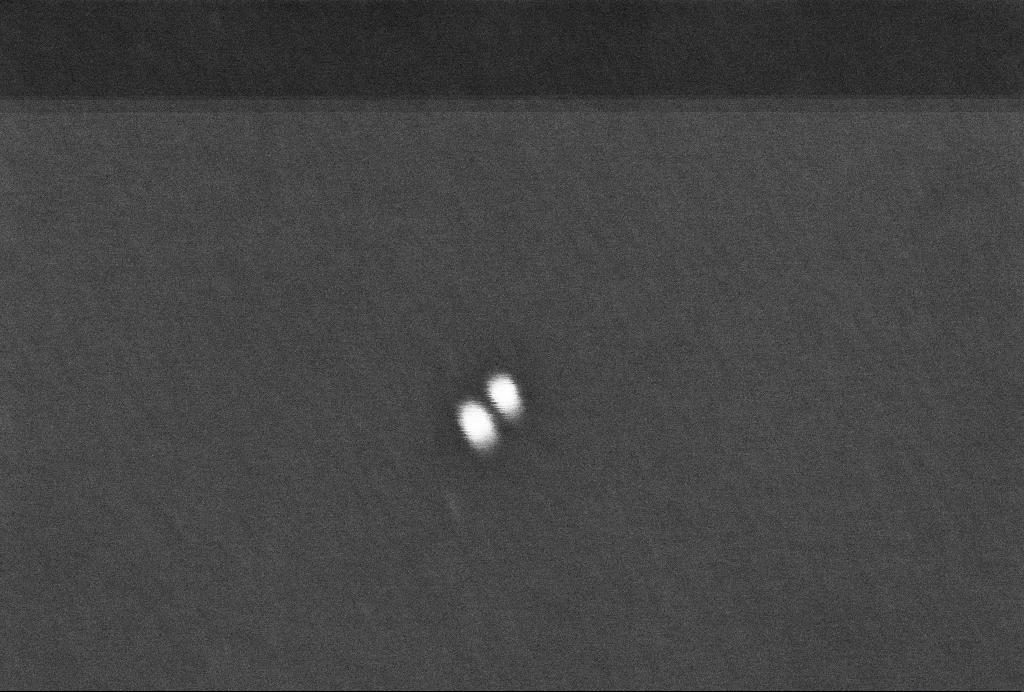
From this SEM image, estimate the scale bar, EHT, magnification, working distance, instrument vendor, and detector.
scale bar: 100 nm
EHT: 2 kV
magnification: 401.53 K X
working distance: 3.3 mm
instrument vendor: Zeiss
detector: InLens